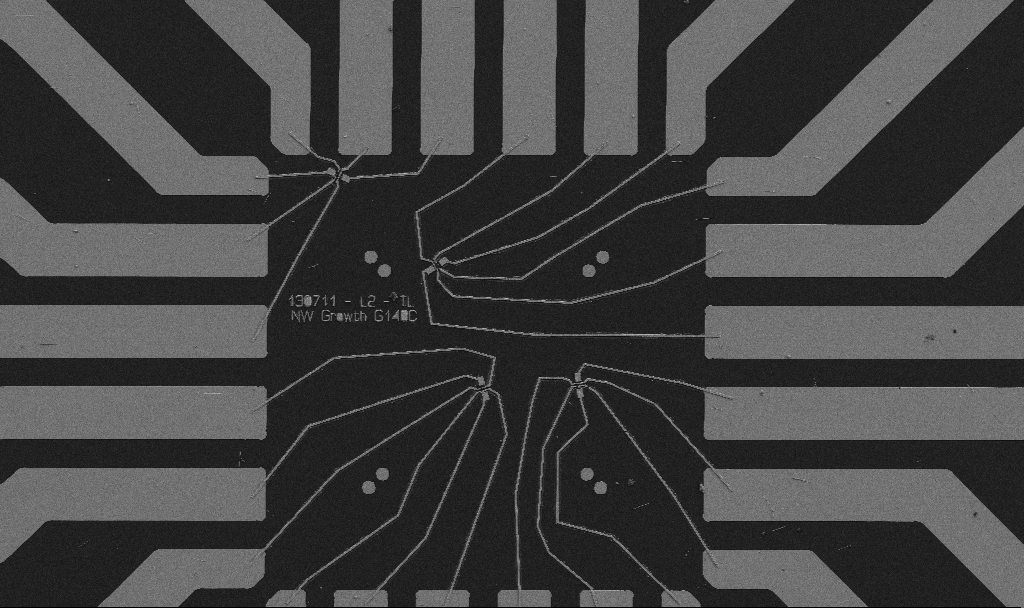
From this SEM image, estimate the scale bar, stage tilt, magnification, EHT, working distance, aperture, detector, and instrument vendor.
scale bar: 20000 nm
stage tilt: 0°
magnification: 1 K X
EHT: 5 kV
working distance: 10.7 mm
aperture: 30 µm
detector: SE2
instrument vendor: Zeiss